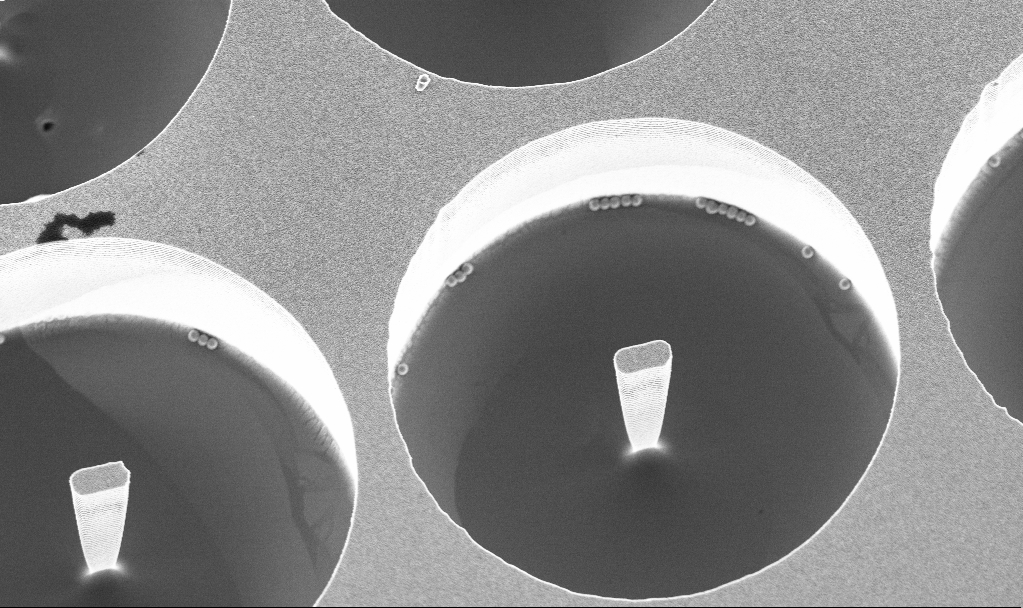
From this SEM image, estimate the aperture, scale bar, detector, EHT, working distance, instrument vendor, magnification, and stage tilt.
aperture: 30 µm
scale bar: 10000 nm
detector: InLens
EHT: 3 kV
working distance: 3.8 mm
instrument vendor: Zeiss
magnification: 6.22 K X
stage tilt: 20°